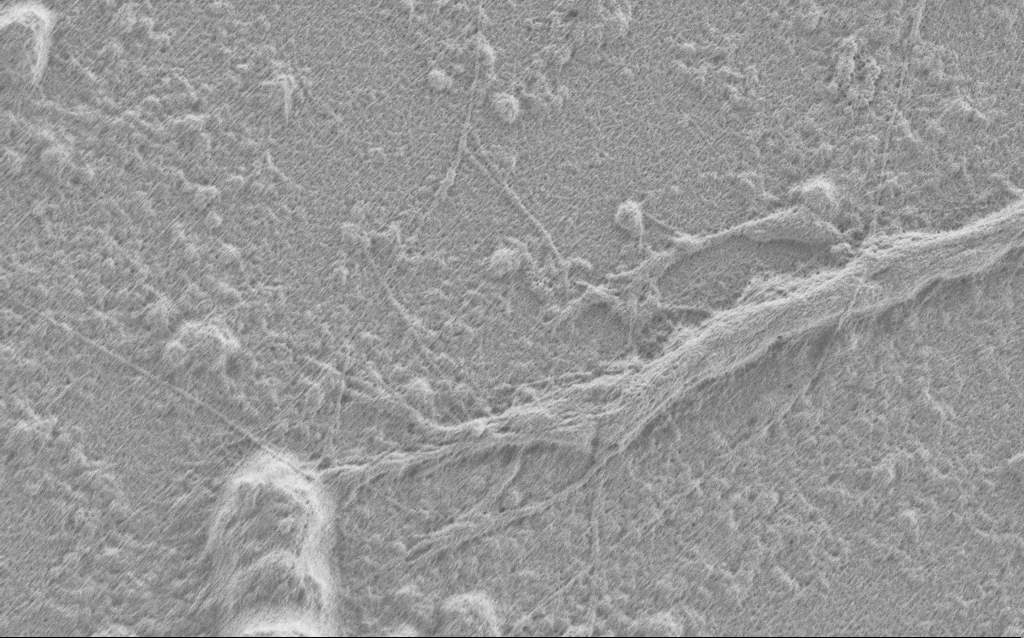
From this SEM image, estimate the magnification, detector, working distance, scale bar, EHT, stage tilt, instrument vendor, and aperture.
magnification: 10 K X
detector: SE2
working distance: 4 mm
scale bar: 2000 nm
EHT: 0.9 kV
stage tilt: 0°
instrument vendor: Zeiss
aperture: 30 µm